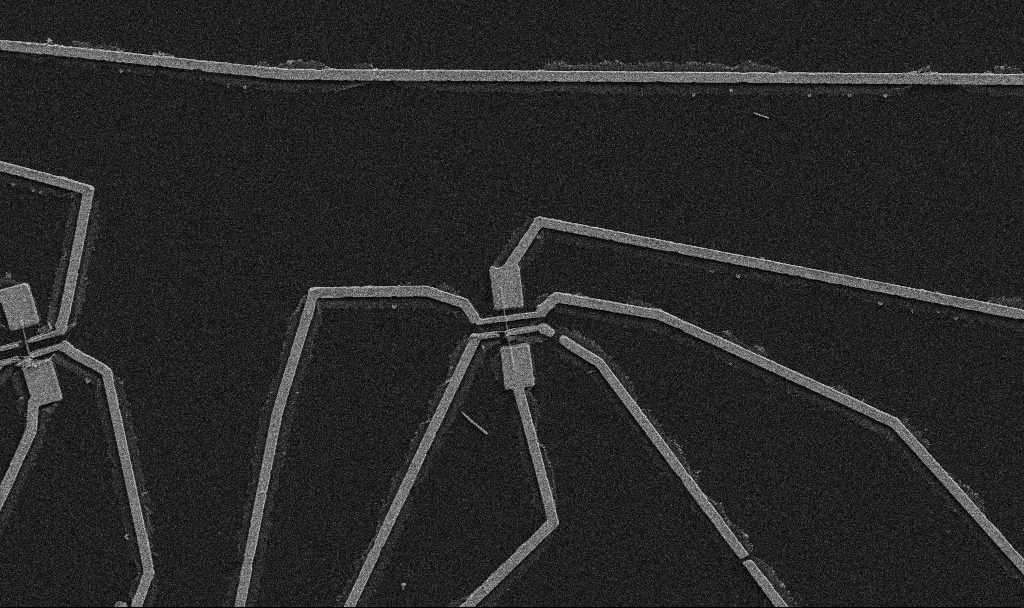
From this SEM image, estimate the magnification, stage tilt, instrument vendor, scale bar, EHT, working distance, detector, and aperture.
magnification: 5 K X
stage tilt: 0°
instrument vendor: Zeiss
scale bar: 10000 nm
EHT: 5 kV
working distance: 10.7 mm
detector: SE2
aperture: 30 µm